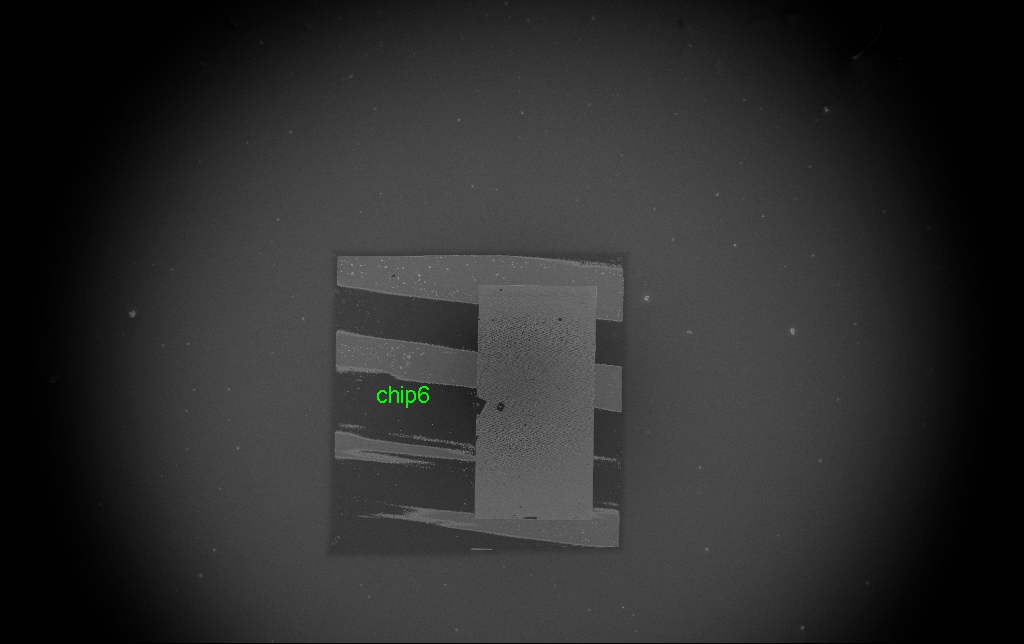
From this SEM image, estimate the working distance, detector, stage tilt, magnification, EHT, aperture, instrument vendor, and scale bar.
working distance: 4.8 mm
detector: InLens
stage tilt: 0°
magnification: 0.087 K X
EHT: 5 kV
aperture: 30 µm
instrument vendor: Zeiss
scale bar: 200000 nm